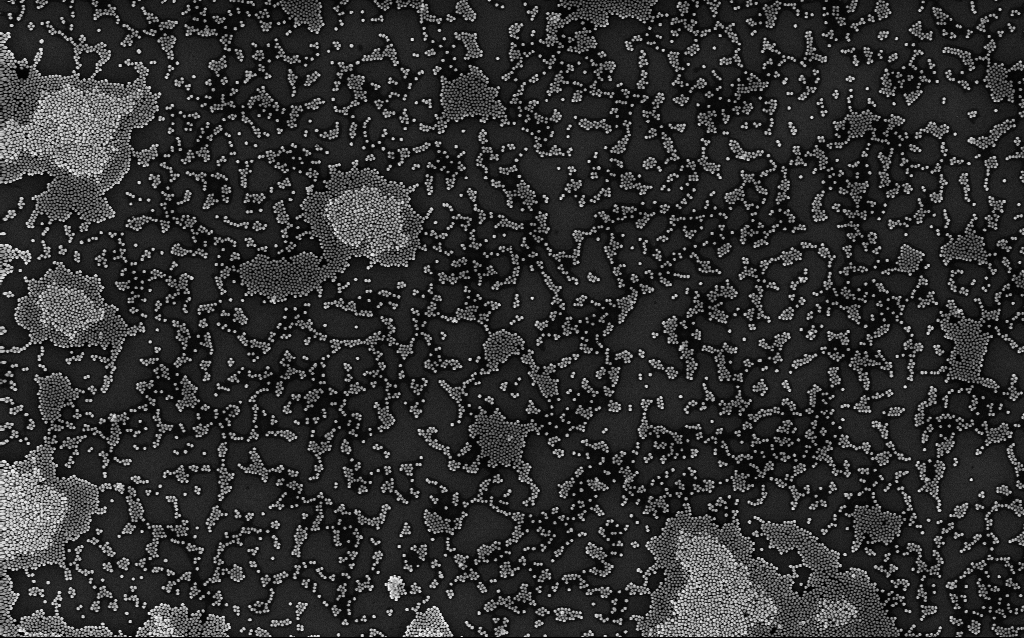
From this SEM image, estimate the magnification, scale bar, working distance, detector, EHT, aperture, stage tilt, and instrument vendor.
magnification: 19.87 K X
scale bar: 1000 nm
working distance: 3.1 mm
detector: InLens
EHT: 8 kV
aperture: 30 µm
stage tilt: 0°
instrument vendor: Zeiss